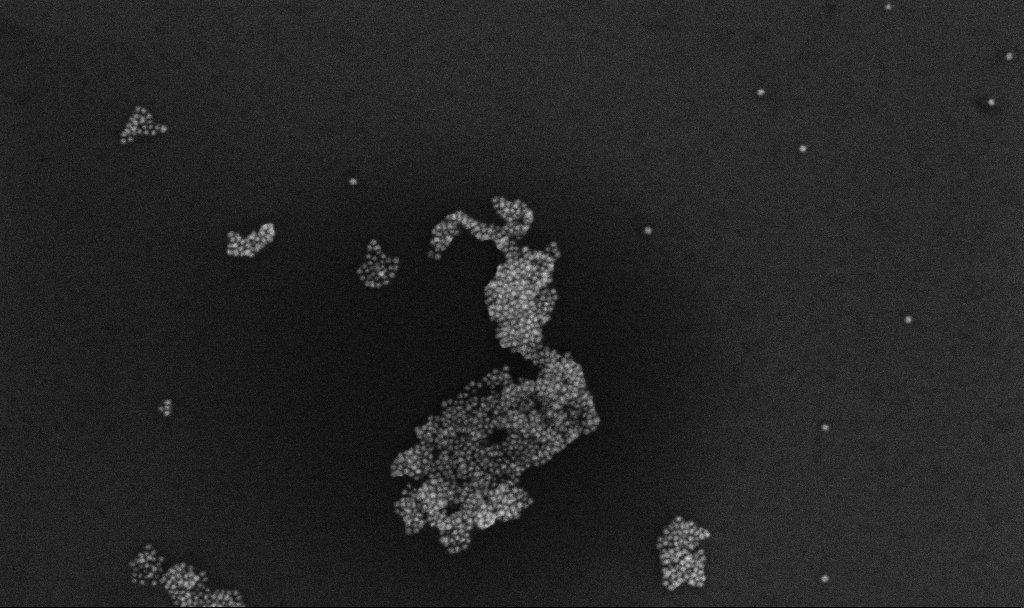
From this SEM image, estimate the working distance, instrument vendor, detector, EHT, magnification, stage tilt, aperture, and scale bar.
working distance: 3.3 mm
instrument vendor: Zeiss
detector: InLens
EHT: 10 kV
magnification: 100 K X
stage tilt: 0°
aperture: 30 µm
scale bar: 200 nm